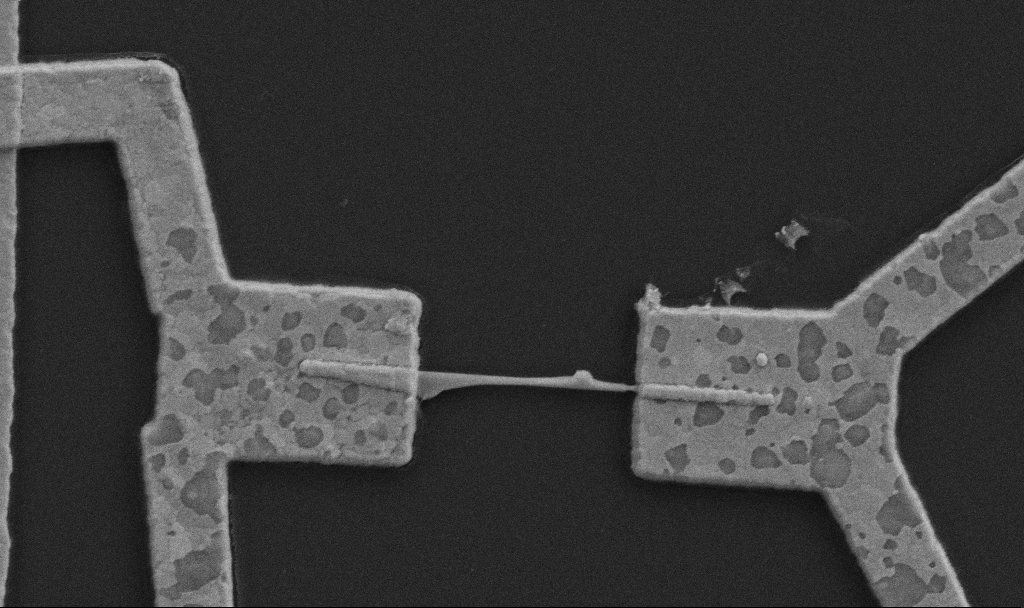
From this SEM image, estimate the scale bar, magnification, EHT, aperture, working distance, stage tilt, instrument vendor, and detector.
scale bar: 1000 nm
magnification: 30 K X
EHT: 5 kV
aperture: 30 µm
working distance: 10.7 mm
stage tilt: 0°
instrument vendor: Zeiss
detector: SE2